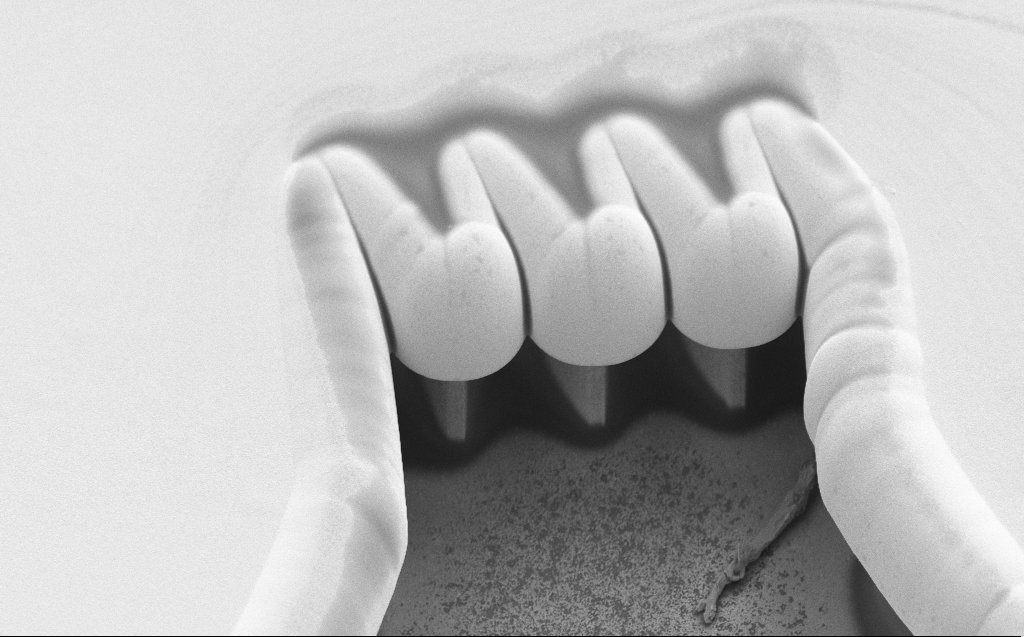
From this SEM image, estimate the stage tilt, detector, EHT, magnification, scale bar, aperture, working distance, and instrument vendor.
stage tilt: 44.4°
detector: SE2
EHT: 5 kV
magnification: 4.54 K X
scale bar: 10000 nm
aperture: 30 µm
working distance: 11 mm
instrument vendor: Zeiss